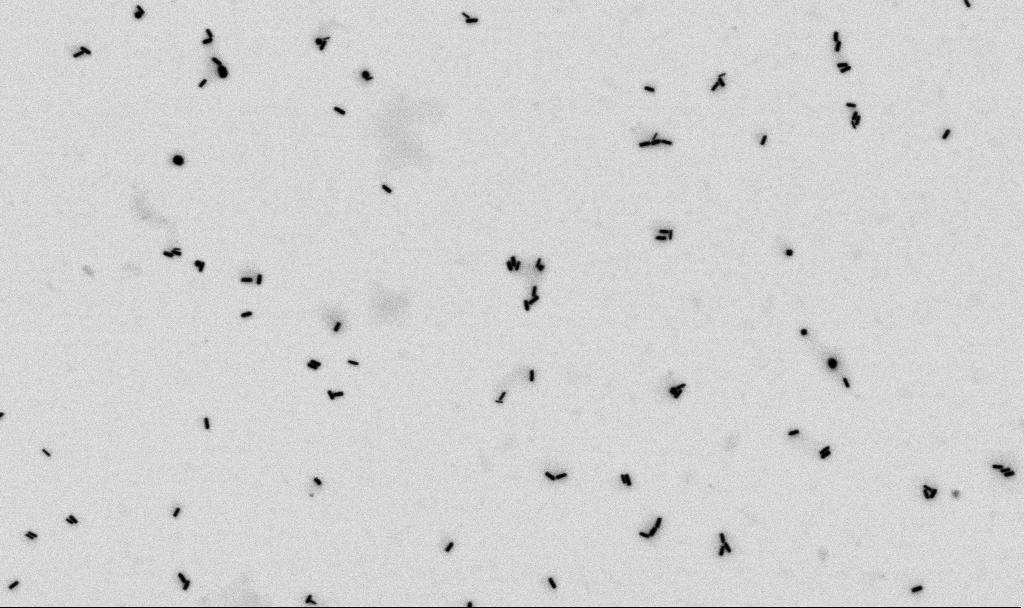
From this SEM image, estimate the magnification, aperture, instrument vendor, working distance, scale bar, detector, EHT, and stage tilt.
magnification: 50 K X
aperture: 30 µm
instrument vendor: Zeiss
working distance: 4.5 mm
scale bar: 1000 nm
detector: SE2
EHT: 2 kV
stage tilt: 0°